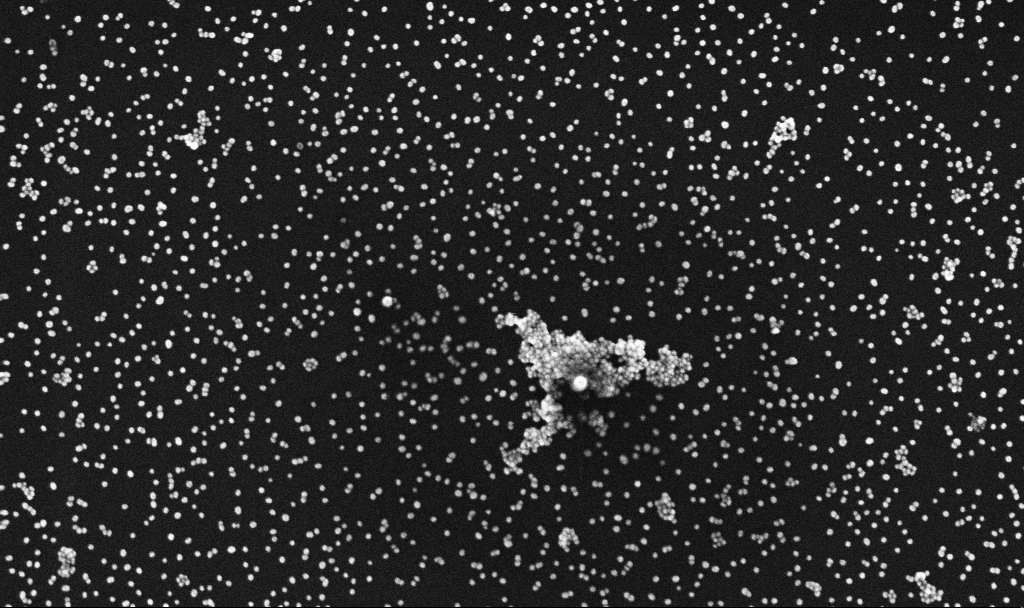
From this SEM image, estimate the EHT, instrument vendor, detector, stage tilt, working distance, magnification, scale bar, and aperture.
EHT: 10 kV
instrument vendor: Zeiss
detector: InLens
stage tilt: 0°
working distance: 5.4 mm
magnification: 100 K X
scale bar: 200 nm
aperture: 30 µm